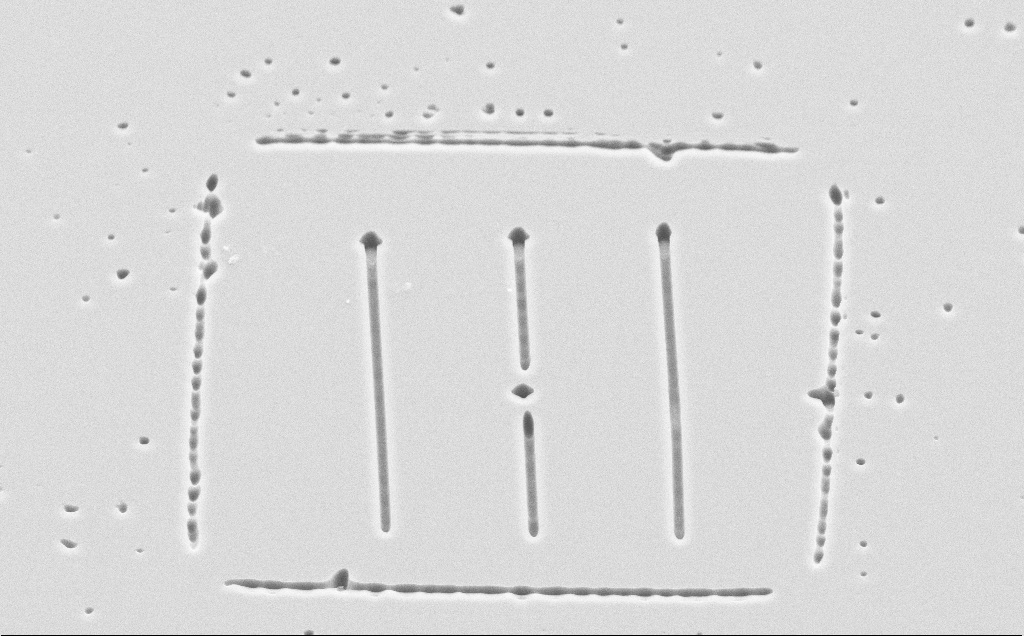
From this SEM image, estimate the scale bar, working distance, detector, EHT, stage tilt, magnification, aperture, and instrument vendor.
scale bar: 10000 nm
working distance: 8 mm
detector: SE2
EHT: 10 kV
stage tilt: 45°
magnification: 2.69 K X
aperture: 30 µm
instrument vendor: Zeiss